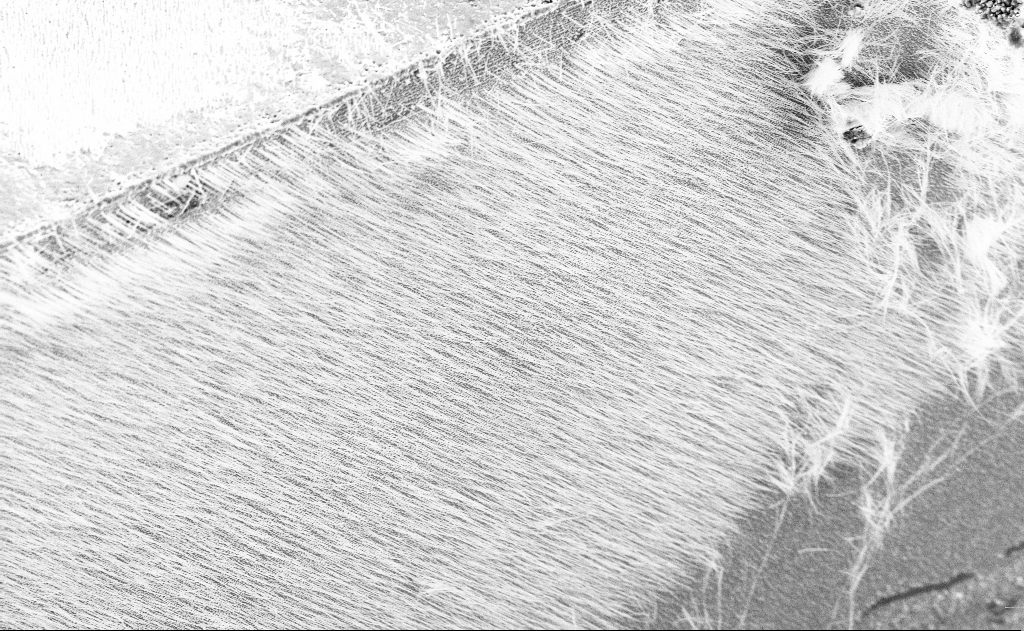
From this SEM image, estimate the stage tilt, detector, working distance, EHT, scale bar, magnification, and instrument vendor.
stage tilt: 45°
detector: SE2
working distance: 14 mm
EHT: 10 kV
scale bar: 10000 nm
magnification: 3.56 K X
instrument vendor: Zeiss